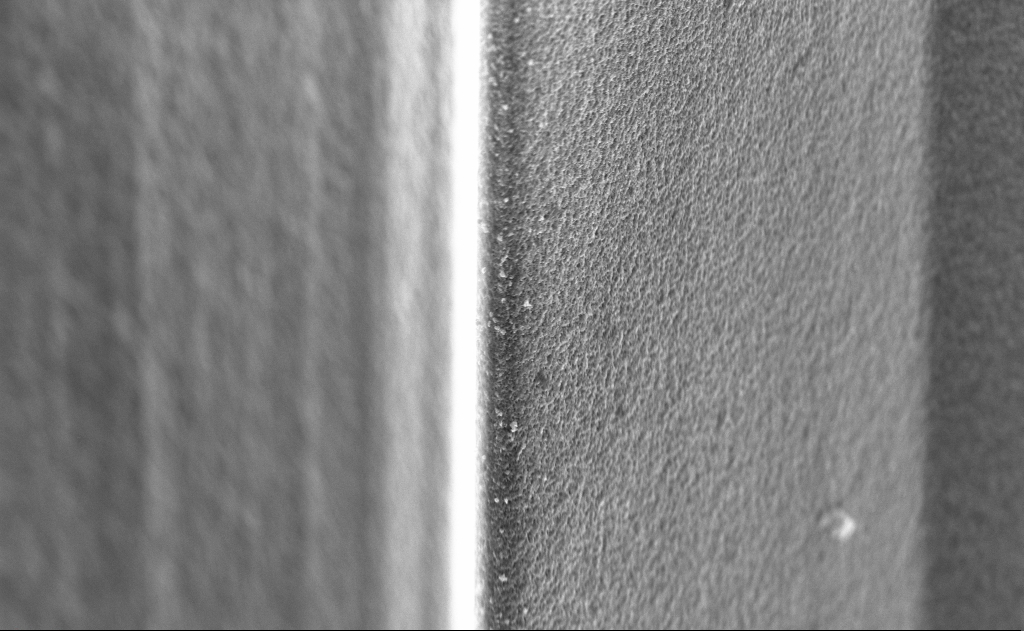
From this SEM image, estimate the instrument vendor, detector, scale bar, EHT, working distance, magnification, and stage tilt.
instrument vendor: Zeiss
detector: InLens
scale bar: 2000 nm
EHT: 10 kV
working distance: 4 mm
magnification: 19.11 K X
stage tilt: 45°